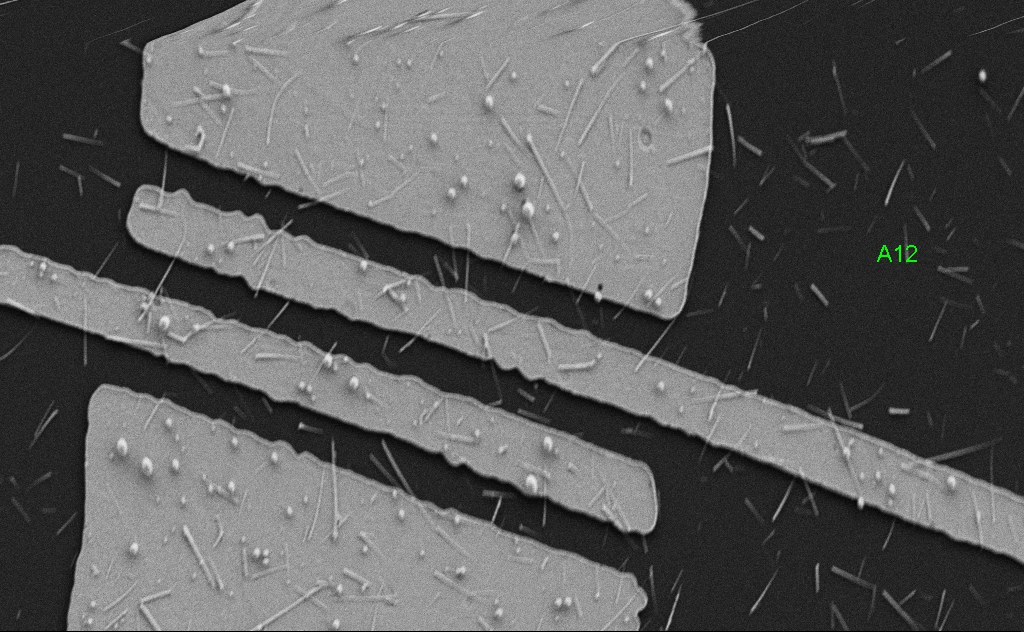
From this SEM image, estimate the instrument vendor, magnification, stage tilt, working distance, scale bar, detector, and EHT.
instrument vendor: Zeiss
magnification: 7.08 K X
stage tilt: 0°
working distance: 5 mm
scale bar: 10000 nm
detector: SE2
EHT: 5 kV